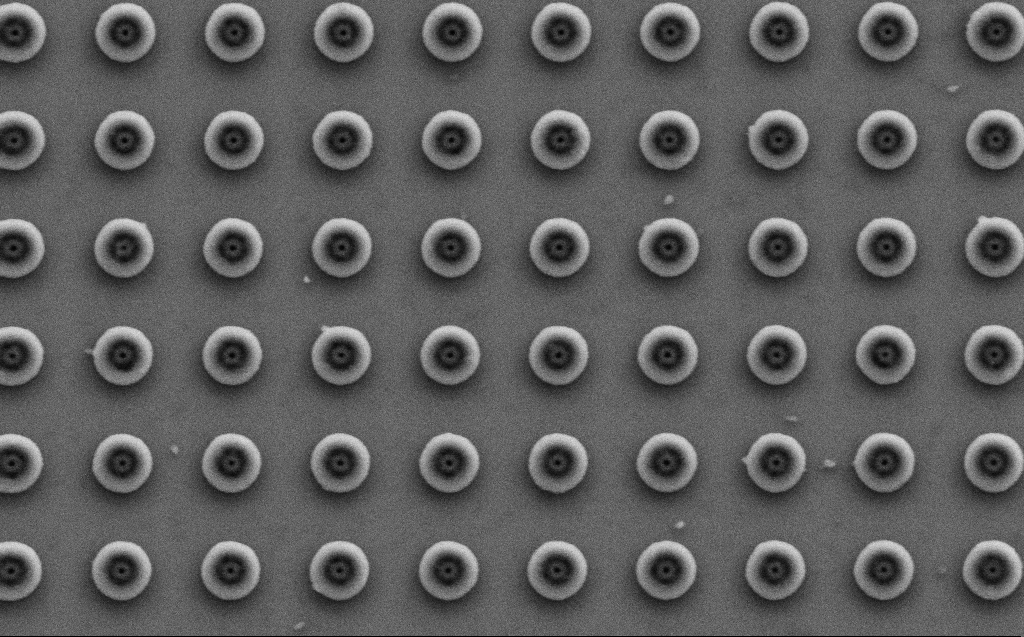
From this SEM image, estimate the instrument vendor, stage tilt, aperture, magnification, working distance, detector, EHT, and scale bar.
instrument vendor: Zeiss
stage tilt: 0°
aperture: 30 µm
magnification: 33.47 K X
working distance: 6 mm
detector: SE2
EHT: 3 kV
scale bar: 2000 nm